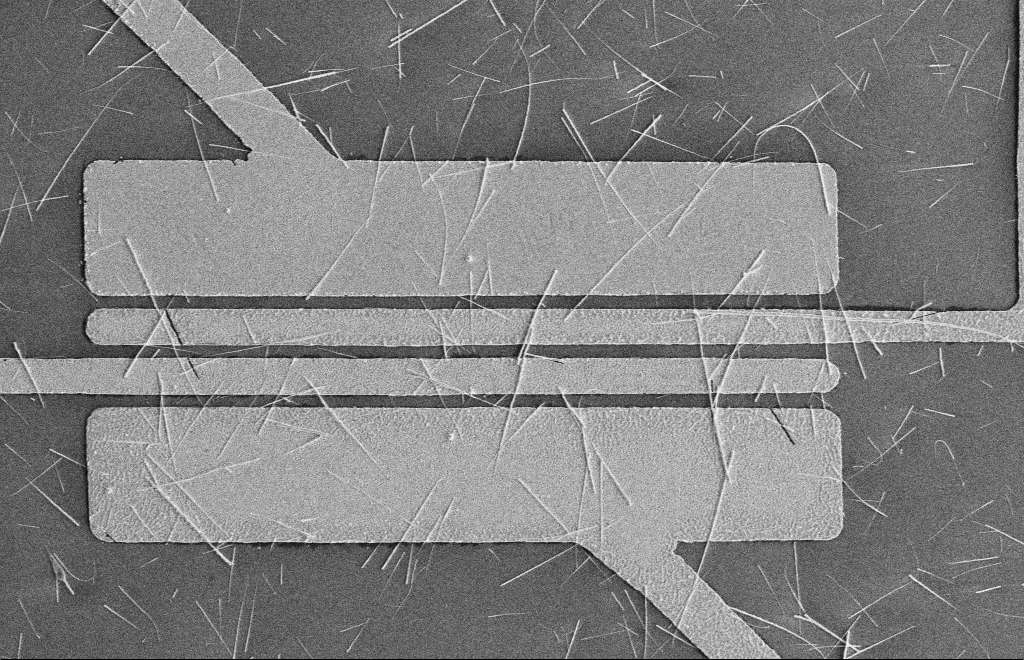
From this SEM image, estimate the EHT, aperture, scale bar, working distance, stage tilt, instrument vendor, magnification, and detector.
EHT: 5 kV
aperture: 10 µm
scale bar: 10000 nm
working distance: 16 mm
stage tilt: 0°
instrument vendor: Zeiss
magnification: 4.56 K X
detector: SE2